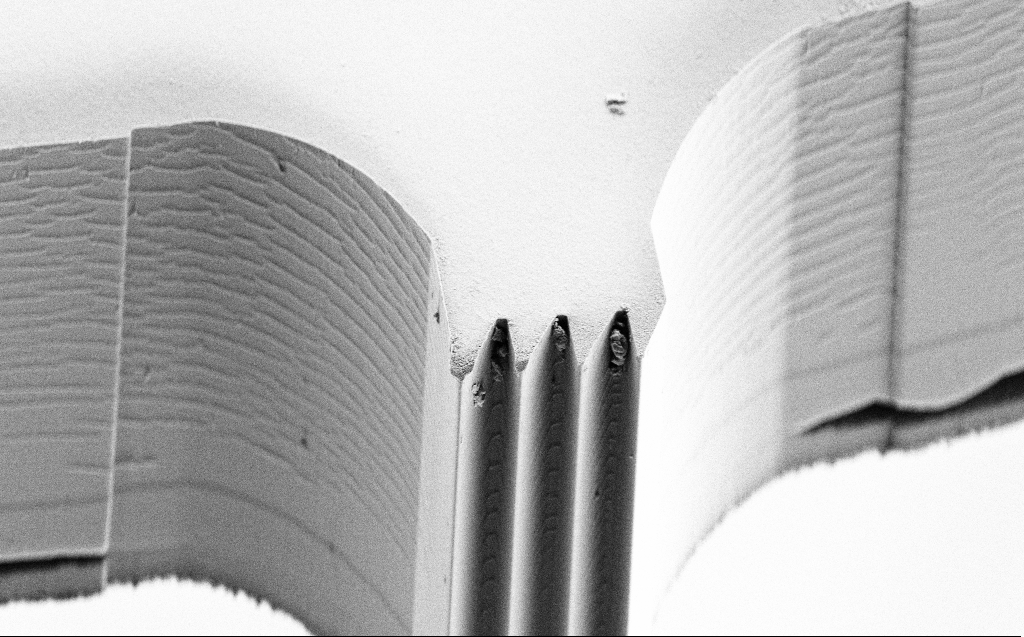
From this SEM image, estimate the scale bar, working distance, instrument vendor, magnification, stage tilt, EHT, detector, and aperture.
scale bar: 10000 nm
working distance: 4 mm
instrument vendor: Zeiss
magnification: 2.01 K X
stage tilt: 45°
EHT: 5 kV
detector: SE2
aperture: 30 µm